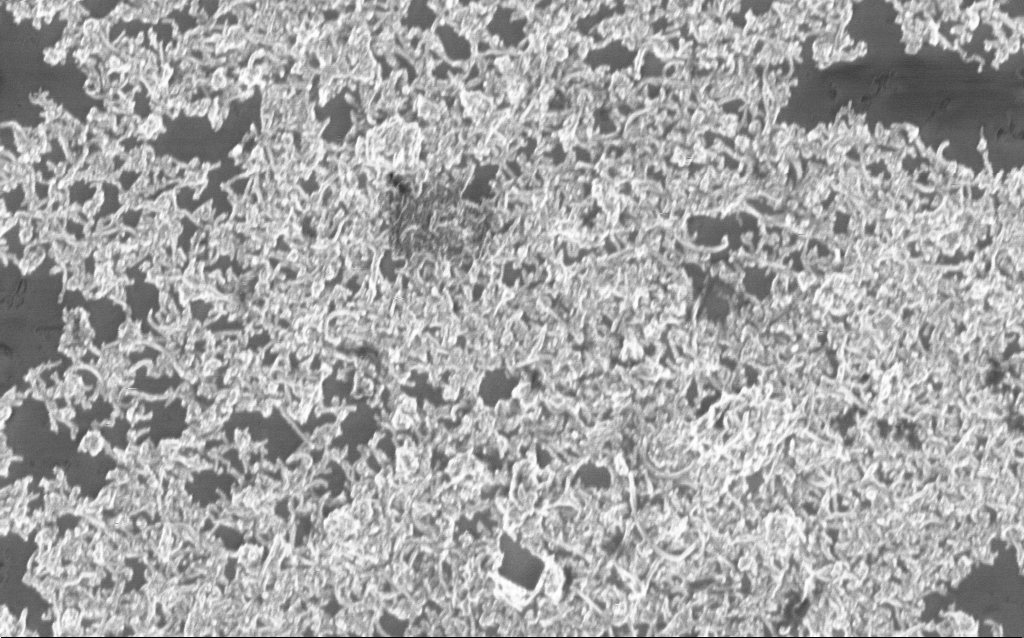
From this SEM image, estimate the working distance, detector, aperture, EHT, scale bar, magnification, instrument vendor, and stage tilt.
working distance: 7 mm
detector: InLens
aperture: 30 µm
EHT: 1 kV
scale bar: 1000 nm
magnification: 25 K X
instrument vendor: Zeiss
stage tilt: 0°